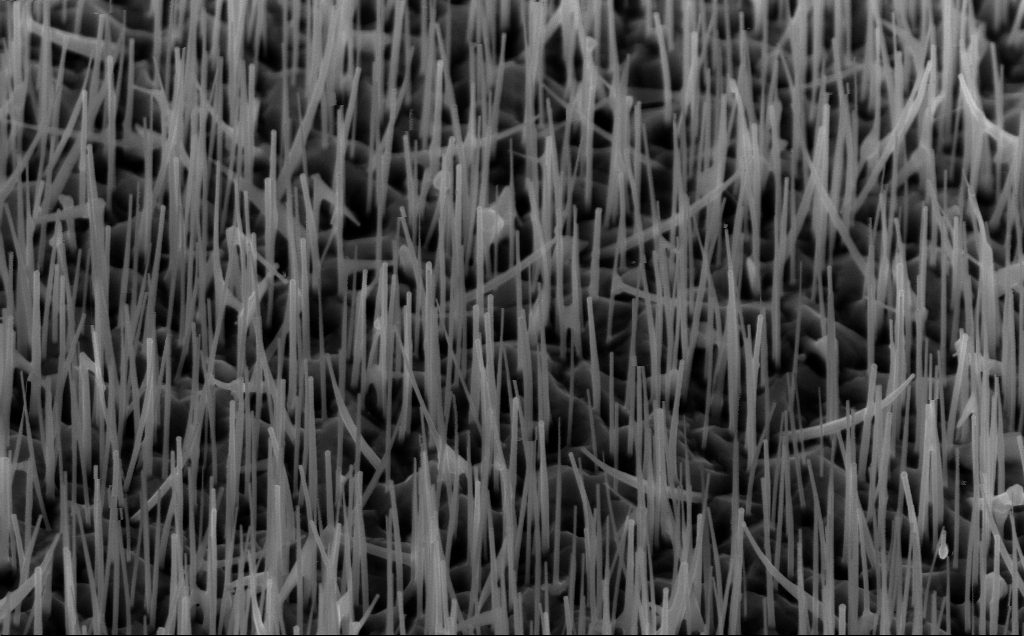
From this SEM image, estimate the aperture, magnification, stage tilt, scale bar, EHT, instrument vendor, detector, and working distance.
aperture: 30 µm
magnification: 40 K X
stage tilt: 45°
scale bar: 1000 nm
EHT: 10 kV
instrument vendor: Zeiss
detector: InLens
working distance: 5 mm